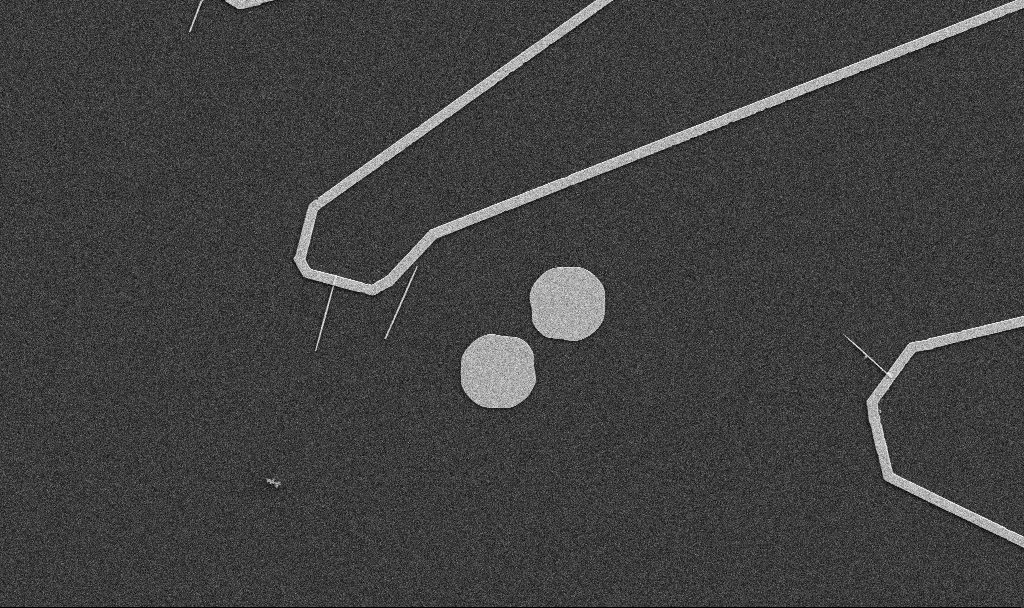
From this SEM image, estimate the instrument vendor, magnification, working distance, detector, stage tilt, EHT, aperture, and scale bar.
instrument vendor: Zeiss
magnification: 5 K X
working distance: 10.7 mm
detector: SE2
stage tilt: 0°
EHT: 5 kV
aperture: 30 µm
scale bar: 10000 nm